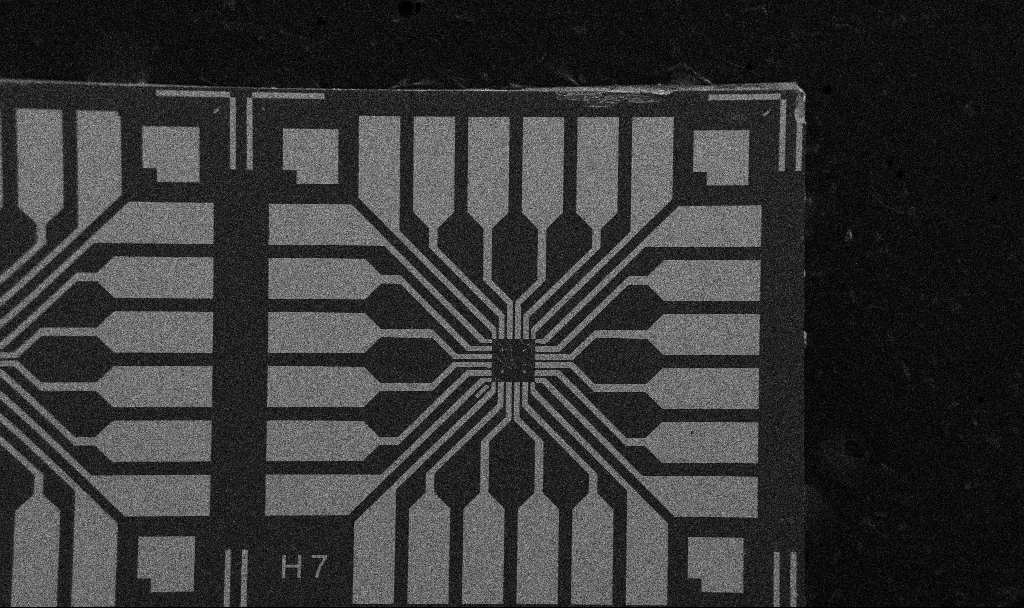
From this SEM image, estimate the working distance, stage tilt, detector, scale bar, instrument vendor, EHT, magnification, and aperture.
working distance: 10.7 mm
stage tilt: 0°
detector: SE2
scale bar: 200000 nm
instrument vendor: Zeiss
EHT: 5 kV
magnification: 0.1 K X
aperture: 30 µm